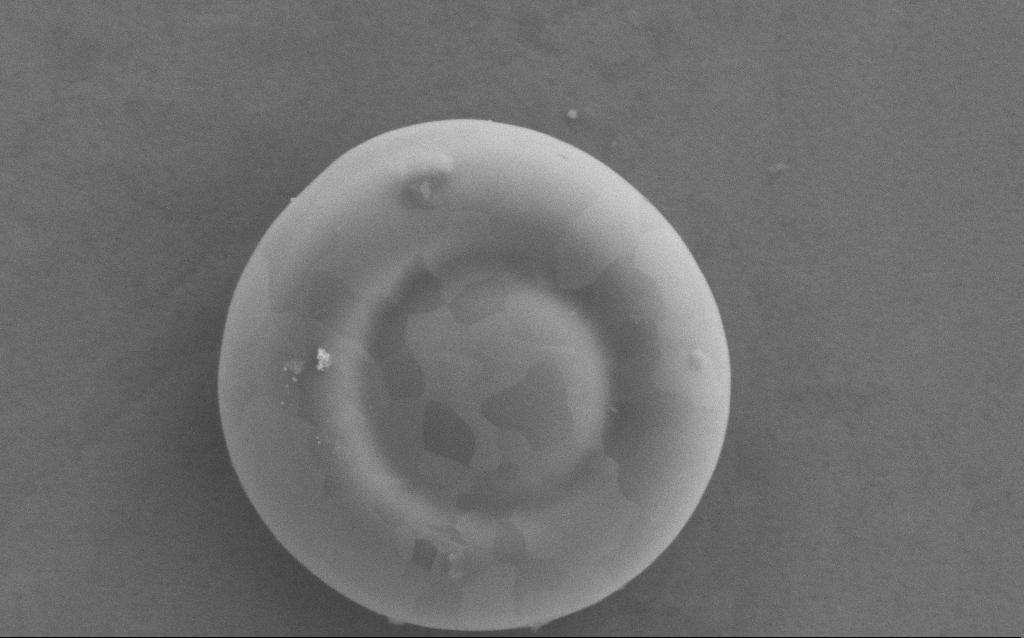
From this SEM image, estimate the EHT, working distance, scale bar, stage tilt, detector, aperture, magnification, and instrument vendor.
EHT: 5 kV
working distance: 4 mm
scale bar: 1000 nm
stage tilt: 0°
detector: SE2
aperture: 30 µm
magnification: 50 K X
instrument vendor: Zeiss